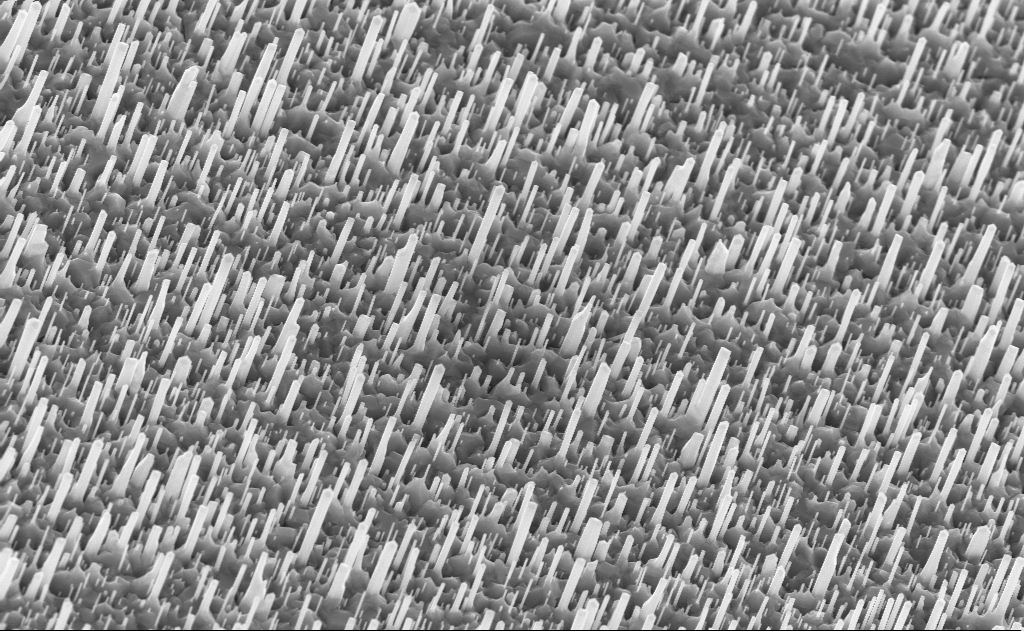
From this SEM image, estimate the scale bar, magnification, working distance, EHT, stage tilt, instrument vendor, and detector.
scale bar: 2000 nm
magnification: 20 K X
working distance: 9 mm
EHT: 10 kV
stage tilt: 0°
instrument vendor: Zeiss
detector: InLens